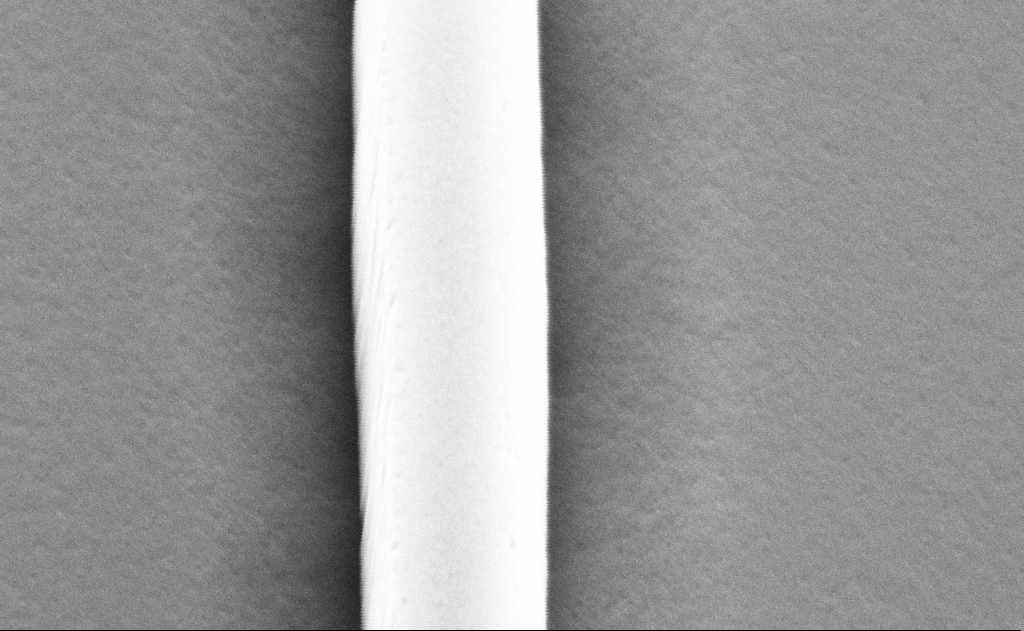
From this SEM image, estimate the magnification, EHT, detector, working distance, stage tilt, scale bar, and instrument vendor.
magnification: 129.25 K X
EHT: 5 kV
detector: SE2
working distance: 11 mm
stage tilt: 45°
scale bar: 200 nm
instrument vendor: Zeiss